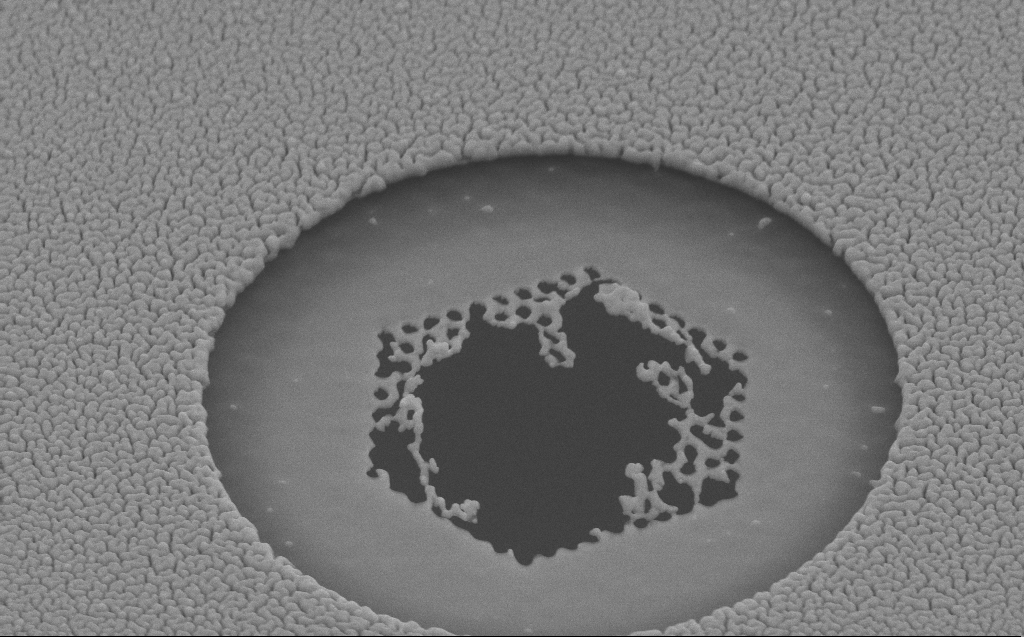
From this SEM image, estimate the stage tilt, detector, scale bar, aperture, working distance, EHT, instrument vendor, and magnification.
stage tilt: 45°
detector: SE2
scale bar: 1000 nm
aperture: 30 µm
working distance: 6 mm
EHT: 10 kV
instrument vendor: Zeiss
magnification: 36.34 K X